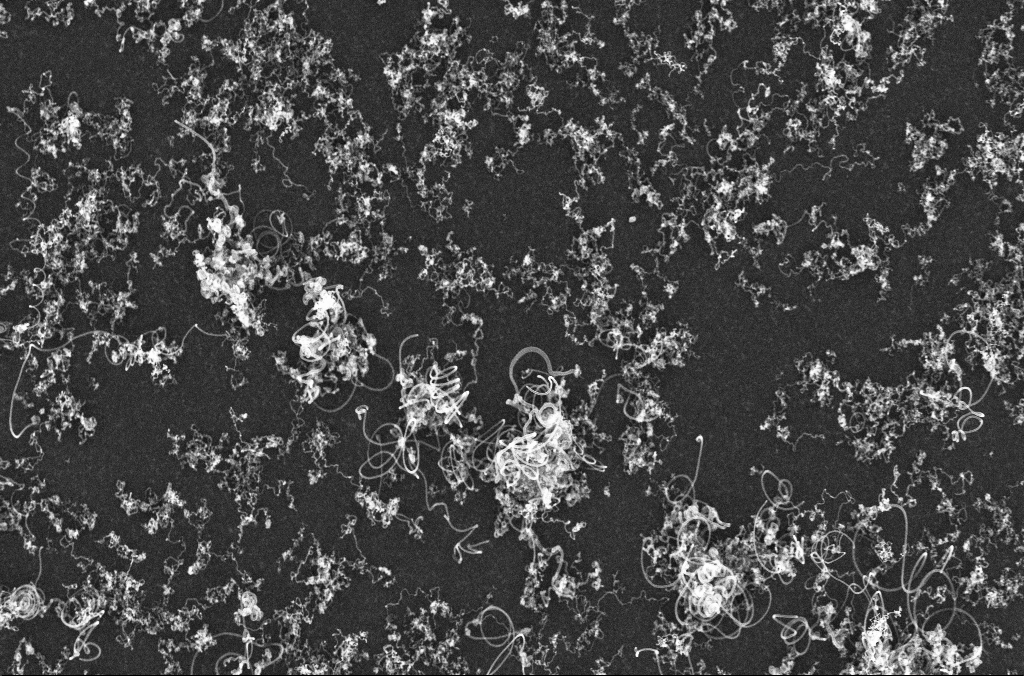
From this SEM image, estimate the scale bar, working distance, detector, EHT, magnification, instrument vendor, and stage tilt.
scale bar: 10000 nm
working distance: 4.4 mm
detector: InLens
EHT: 20 kV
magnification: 5 K X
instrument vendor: Zeiss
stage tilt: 0°